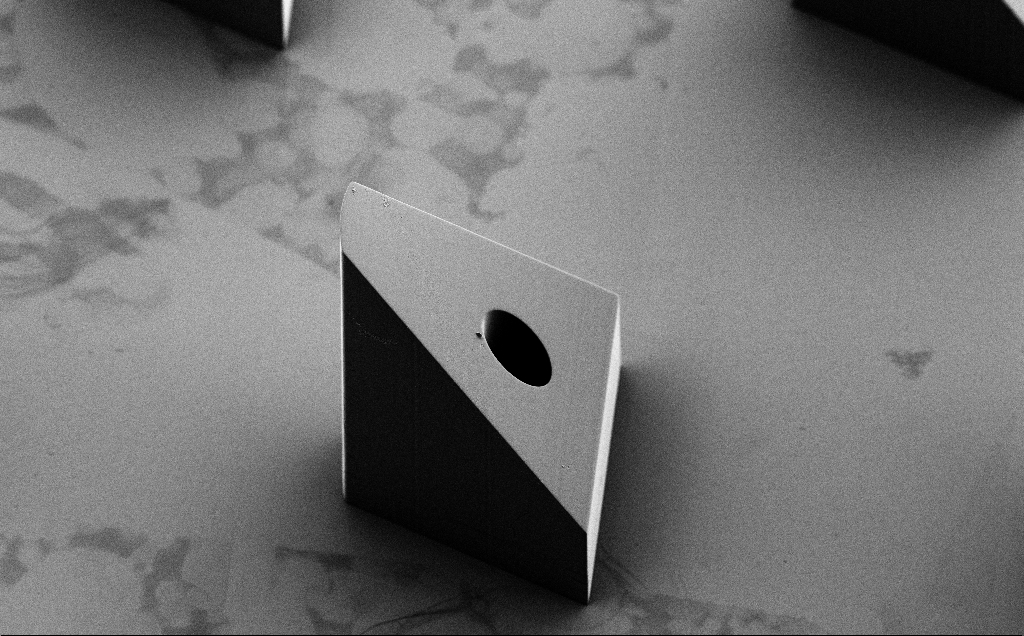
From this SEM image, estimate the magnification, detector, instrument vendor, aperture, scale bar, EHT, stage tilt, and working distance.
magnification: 0.284 K X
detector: SE2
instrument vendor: Zeiss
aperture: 30 µm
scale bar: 200000 nm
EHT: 5 kV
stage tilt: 35°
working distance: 8 mm